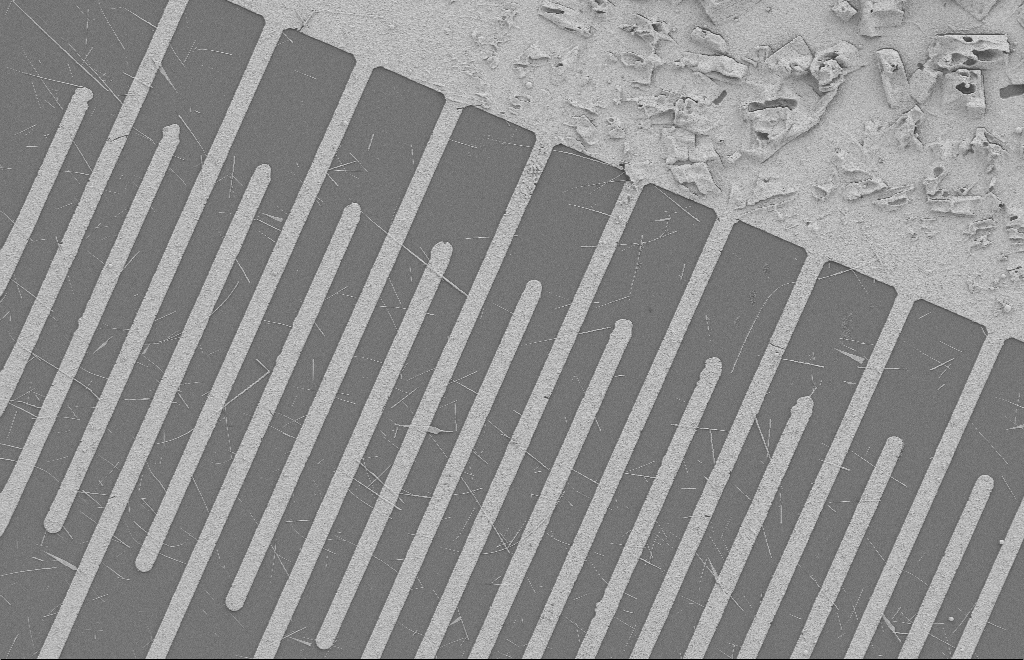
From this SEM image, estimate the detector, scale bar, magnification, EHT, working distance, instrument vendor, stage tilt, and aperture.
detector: SE2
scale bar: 10000 nm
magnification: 2.57 K X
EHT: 2 kV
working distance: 8 mm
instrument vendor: Zeiss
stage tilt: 0°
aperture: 20 µm